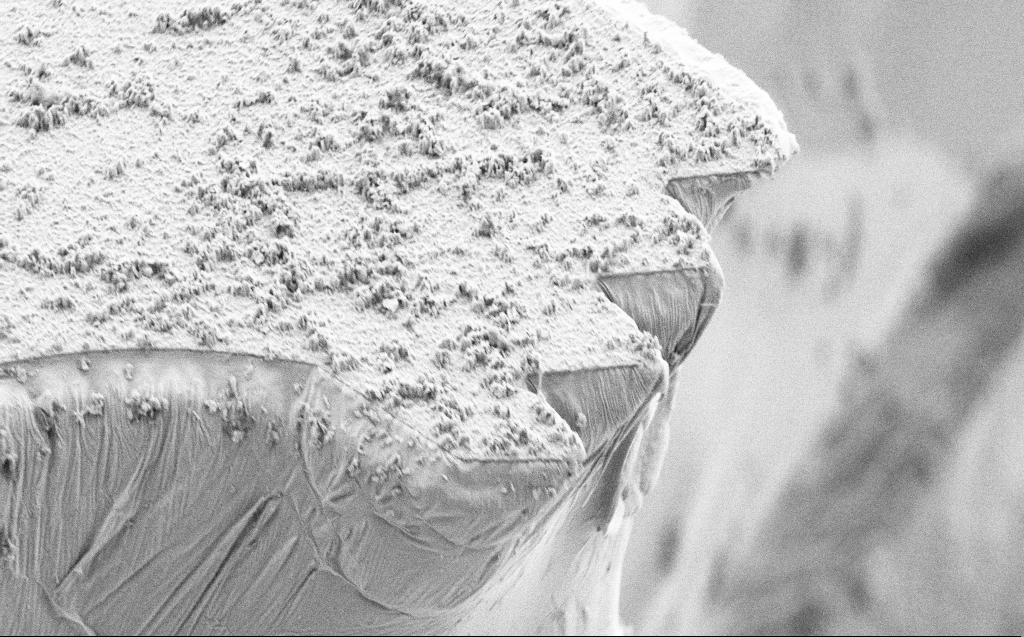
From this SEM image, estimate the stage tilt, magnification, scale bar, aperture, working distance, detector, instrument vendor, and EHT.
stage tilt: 45°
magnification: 3.99 K X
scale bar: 10000 nm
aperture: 30 µm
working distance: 9 mm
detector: SE2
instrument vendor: Zeiss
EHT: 5 kV